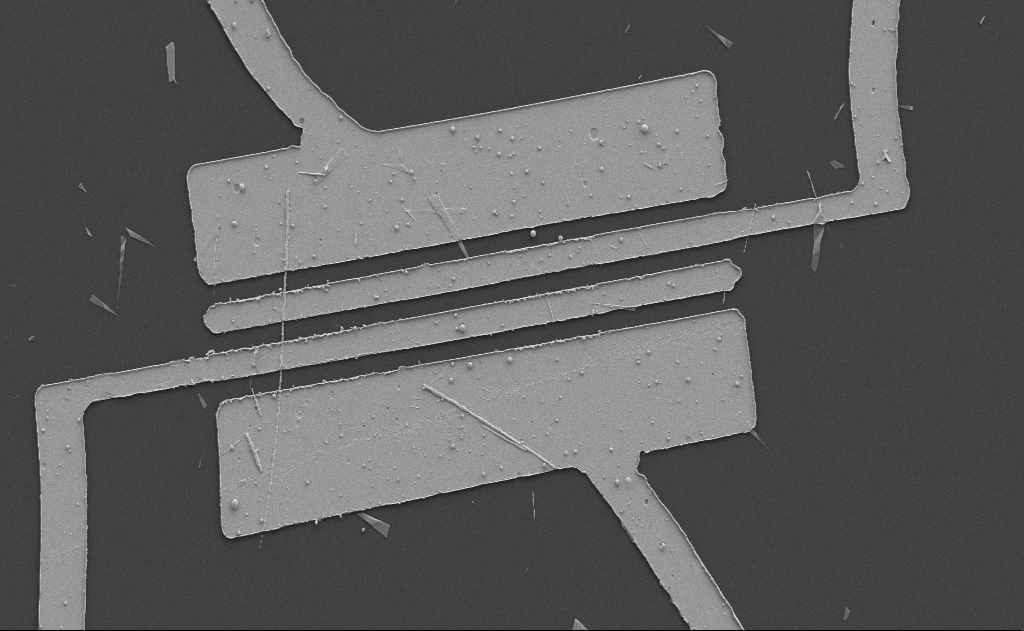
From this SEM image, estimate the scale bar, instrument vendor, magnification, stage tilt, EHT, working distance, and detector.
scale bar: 10000 nm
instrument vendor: Zeiss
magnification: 3.39 K X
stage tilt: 0°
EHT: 5 kV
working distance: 10 mm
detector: SE2